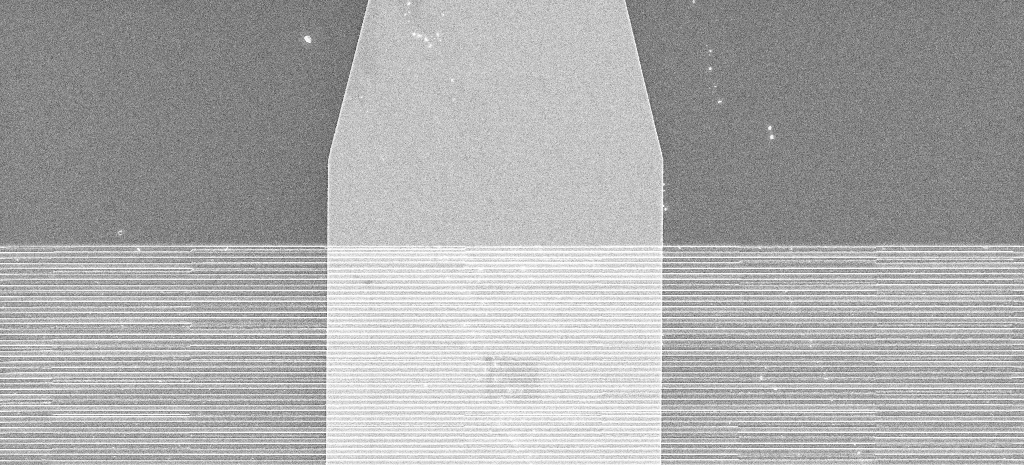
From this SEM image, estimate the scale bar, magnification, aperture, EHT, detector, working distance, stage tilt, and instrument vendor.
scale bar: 10000 nm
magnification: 6.14 K X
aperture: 30 µm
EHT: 5 kV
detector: InLens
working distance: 10.3 mm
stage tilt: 0°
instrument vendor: Zeiss